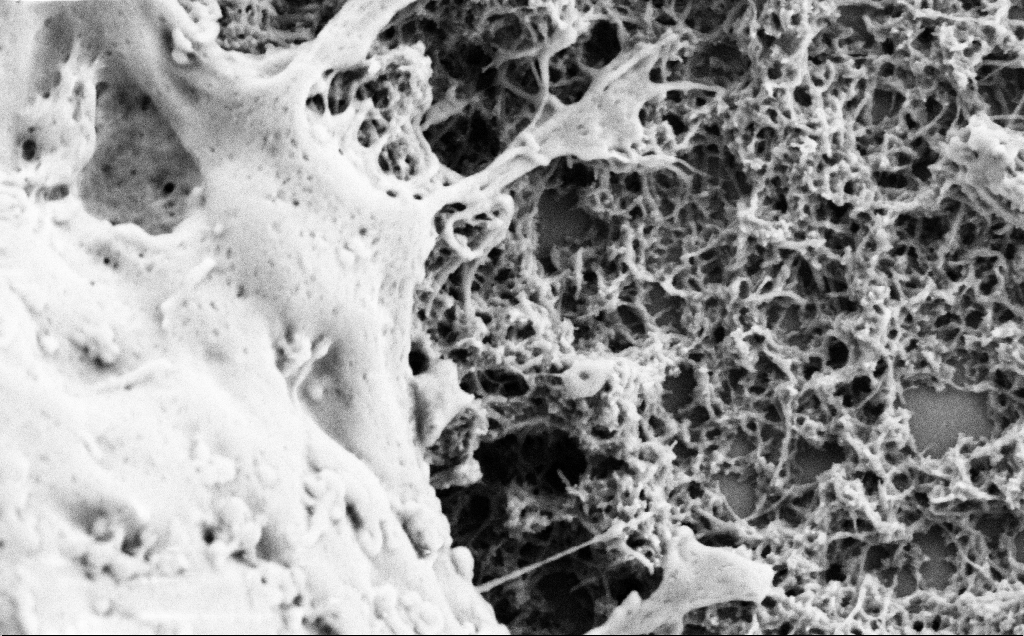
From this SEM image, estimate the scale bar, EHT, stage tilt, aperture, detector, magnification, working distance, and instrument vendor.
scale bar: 1000 nm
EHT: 2 kV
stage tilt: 0°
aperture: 30 µm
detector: SE2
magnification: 50 K X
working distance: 7 mm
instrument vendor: Zeiss